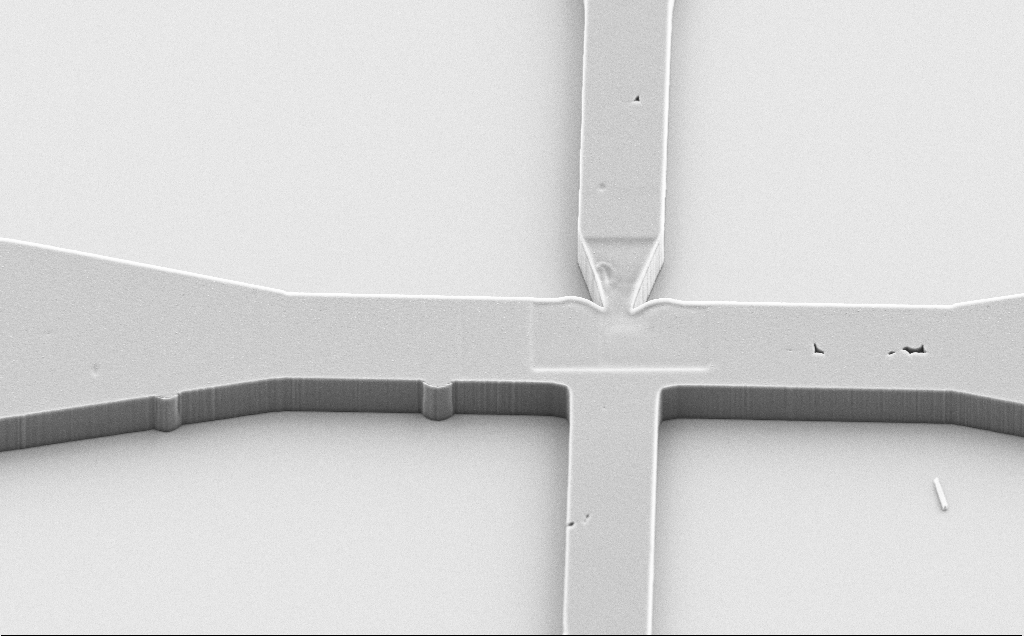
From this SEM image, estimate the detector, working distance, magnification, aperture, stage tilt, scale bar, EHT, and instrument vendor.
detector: SE2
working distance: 9 mm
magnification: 3.11 K X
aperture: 30 µm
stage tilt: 45°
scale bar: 20000 nm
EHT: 5 kV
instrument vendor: Zeiss